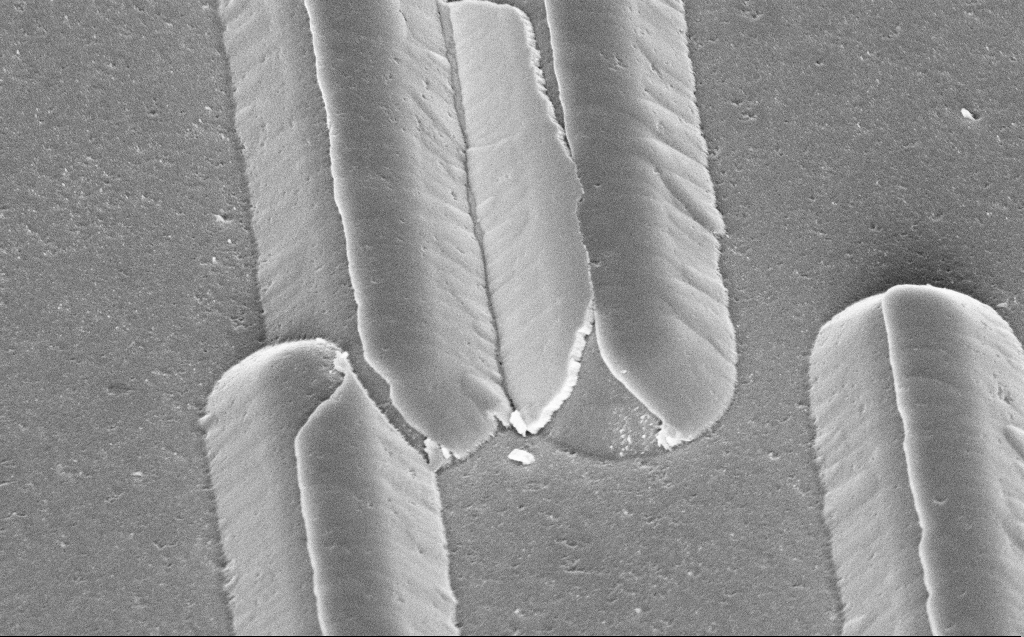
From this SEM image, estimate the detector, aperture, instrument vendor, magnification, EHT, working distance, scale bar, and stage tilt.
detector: InLens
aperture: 30 µm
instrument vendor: Zeiss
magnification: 18.19 K X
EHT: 5 kV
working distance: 11 mm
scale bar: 2000 nm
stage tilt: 45°